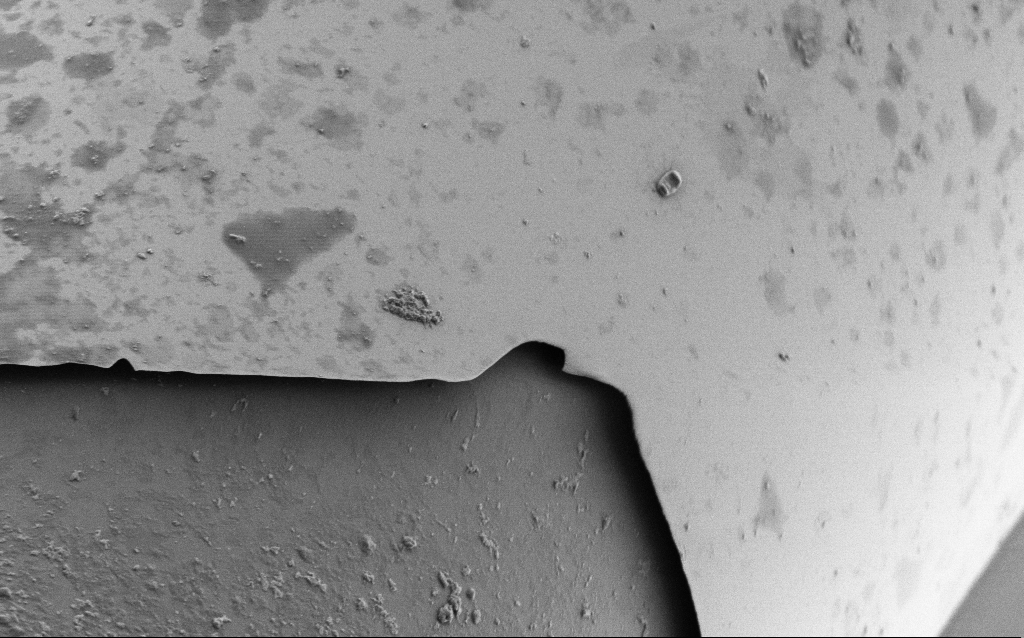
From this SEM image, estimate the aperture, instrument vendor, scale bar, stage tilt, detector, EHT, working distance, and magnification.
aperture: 30 µm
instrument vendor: Zeiss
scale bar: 2000 nm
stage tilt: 45°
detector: SE2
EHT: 1 kV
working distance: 7.7 mm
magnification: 10 K X